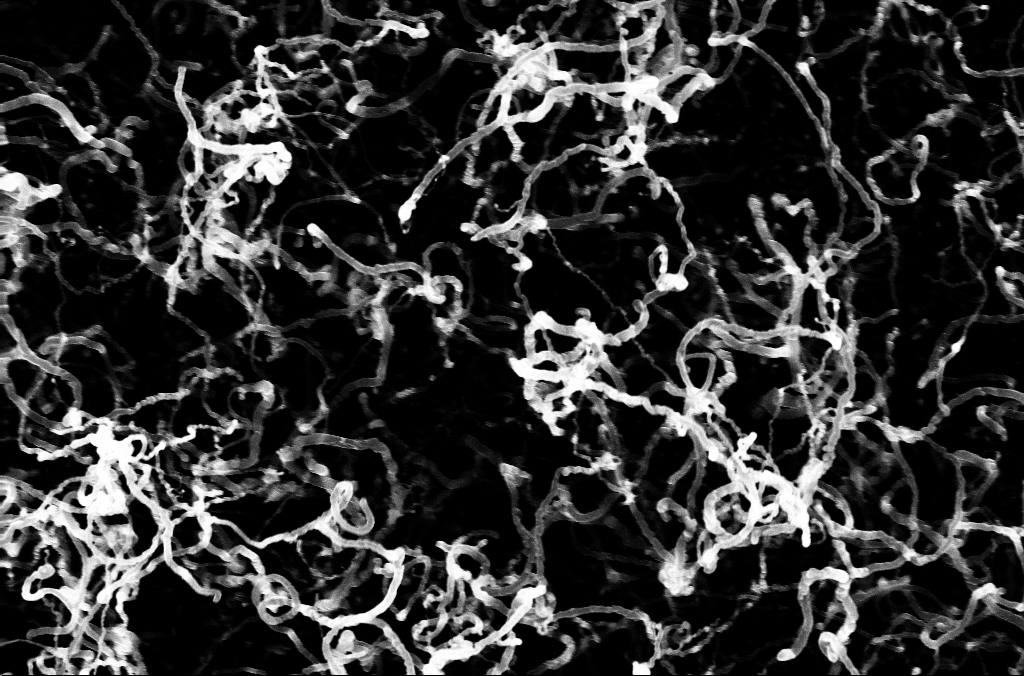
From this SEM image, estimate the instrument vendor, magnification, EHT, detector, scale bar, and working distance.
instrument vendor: Zeiss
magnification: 27.17 K X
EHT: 10 kV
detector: InLens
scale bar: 2000 nm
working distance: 3.2 mm